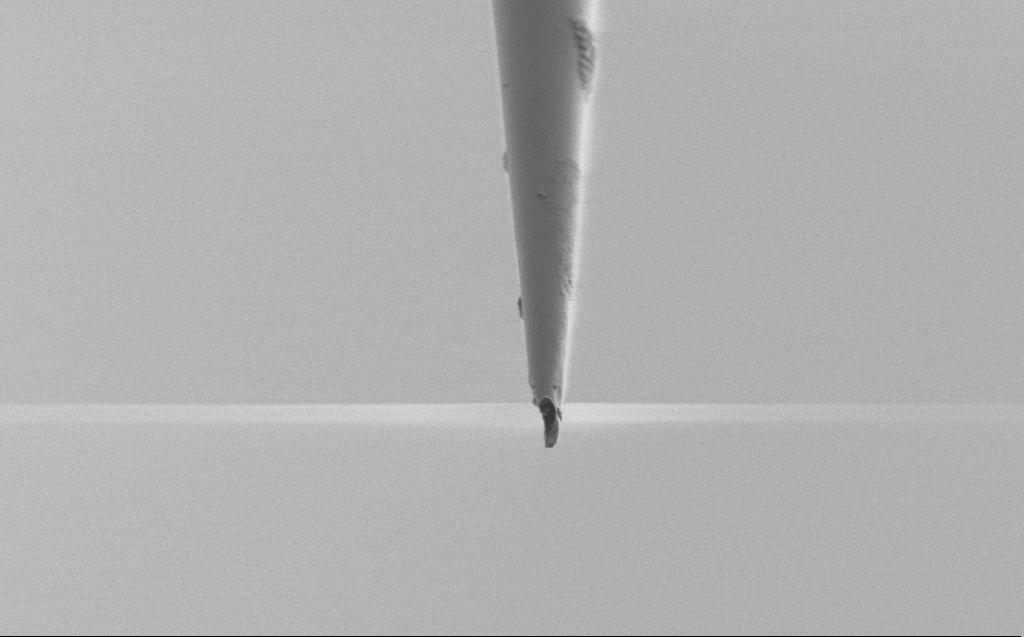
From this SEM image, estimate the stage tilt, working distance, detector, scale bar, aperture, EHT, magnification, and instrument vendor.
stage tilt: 45°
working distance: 5 mm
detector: SE2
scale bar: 2000 nm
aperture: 30 µm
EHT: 1 kV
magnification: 10 K X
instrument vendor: Zeiss